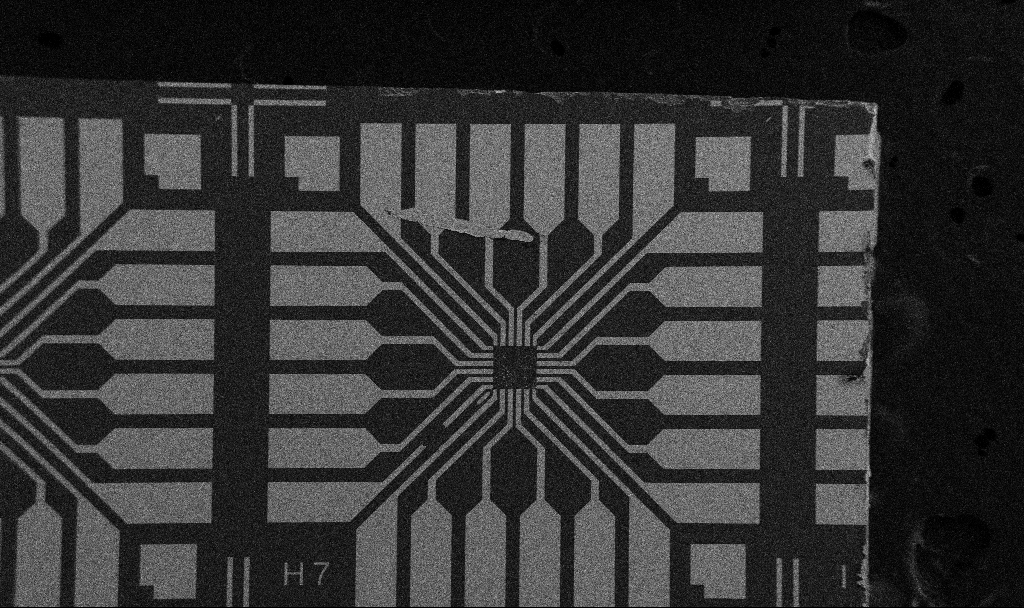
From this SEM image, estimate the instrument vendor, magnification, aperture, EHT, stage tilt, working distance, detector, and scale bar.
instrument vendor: Zeiss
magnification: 0.1 K X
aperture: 30 µm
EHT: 5 kV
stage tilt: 0°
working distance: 10.7 mm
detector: SE2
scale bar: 200000 nm